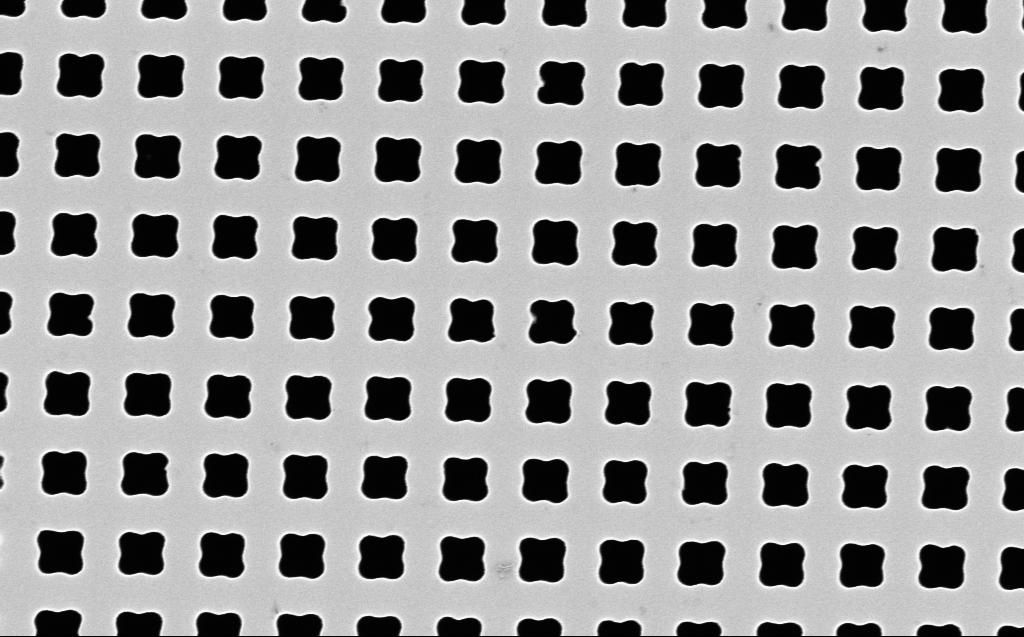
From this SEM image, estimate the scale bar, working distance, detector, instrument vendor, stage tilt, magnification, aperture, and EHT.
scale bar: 1000 nm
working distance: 7 mm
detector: InLens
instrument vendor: Zeiss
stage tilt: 0°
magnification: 59.3 K X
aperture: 30 µm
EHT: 10 kV